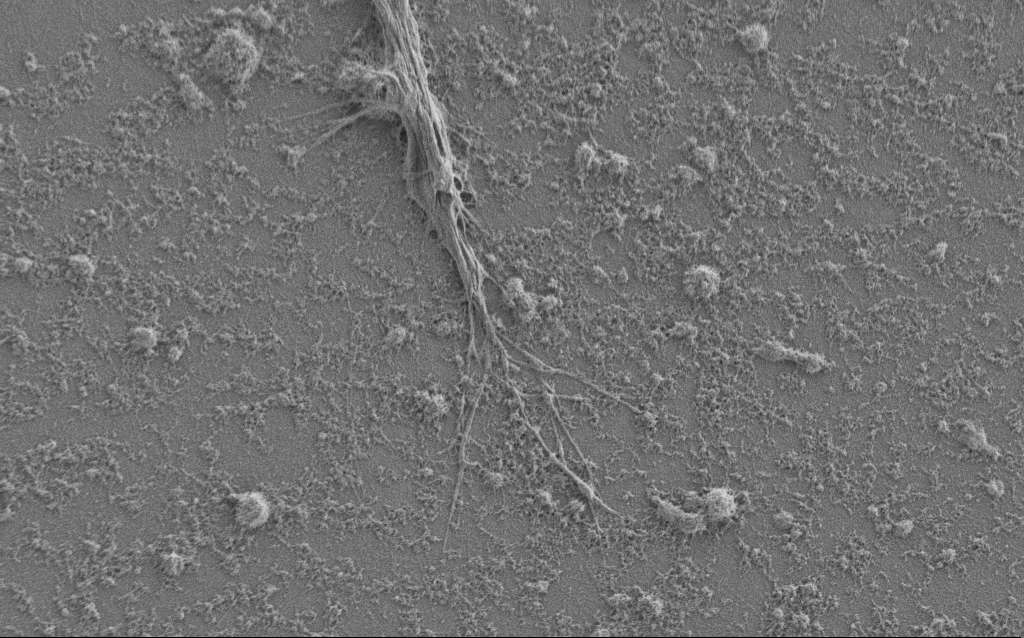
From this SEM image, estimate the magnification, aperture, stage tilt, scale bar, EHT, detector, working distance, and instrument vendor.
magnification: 6 K X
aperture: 30 µm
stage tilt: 0°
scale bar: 10000 nm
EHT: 1 kV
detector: SE2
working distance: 6 mm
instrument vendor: Zeiss